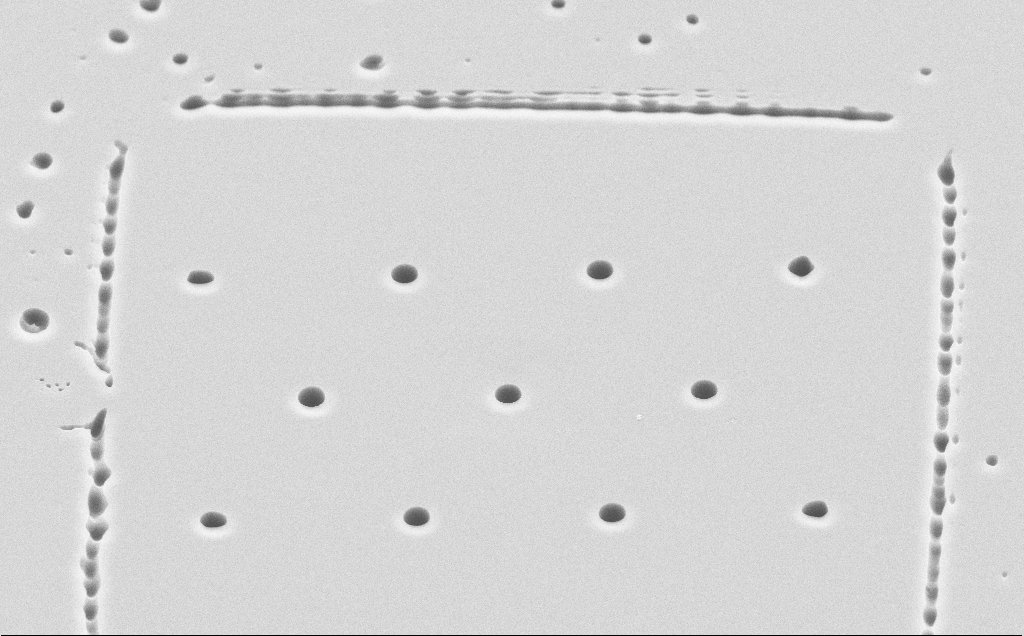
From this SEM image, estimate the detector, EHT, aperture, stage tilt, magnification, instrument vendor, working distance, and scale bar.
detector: SE2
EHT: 10 kV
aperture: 30 µm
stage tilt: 45°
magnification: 3.58 K X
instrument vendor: Zeiss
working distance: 7 mm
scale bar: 10000 nm